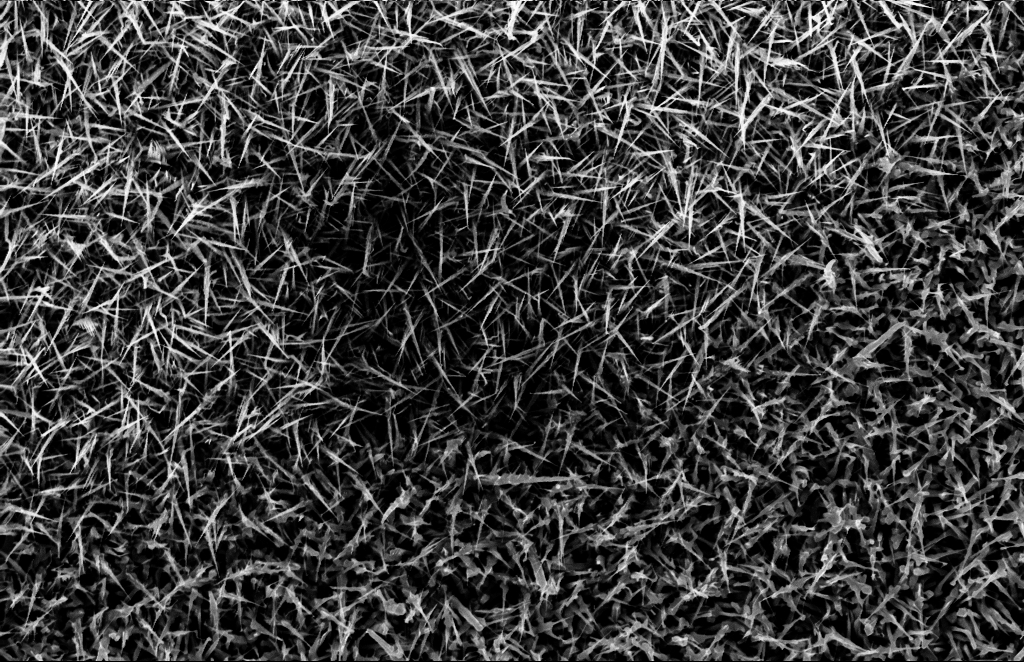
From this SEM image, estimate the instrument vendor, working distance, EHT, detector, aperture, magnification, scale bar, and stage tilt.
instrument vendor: Zeiss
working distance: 10 mm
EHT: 10 kV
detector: InLens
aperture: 30 µm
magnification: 20 K X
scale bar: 2000 nm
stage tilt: -1.1°